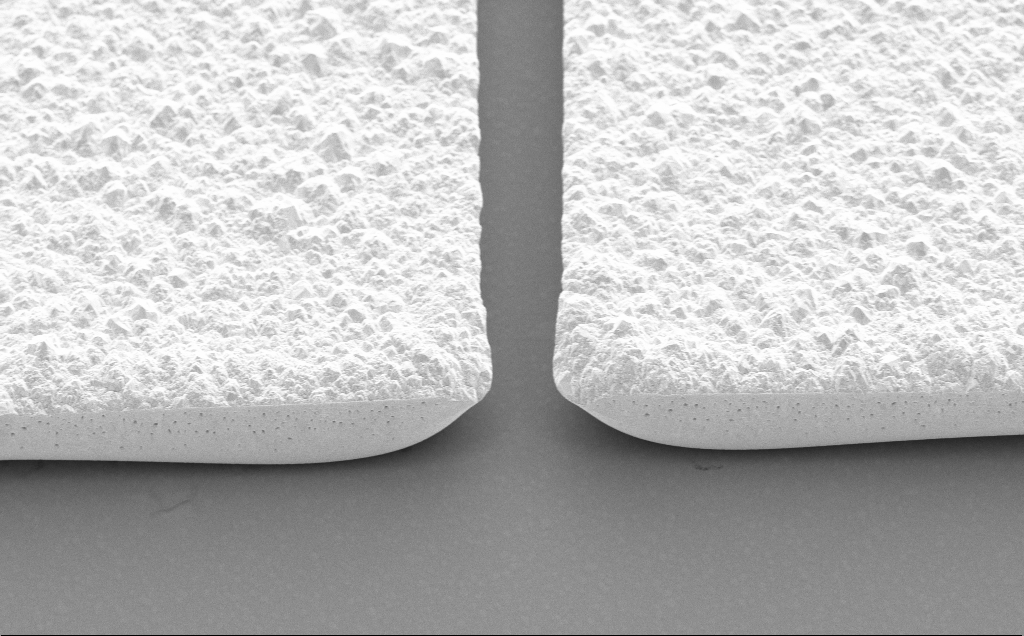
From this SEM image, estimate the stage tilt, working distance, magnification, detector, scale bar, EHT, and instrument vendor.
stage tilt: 45°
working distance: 14 mm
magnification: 3.6 K X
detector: SE2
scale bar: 20000 nm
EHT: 5 kV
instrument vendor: Zeiss